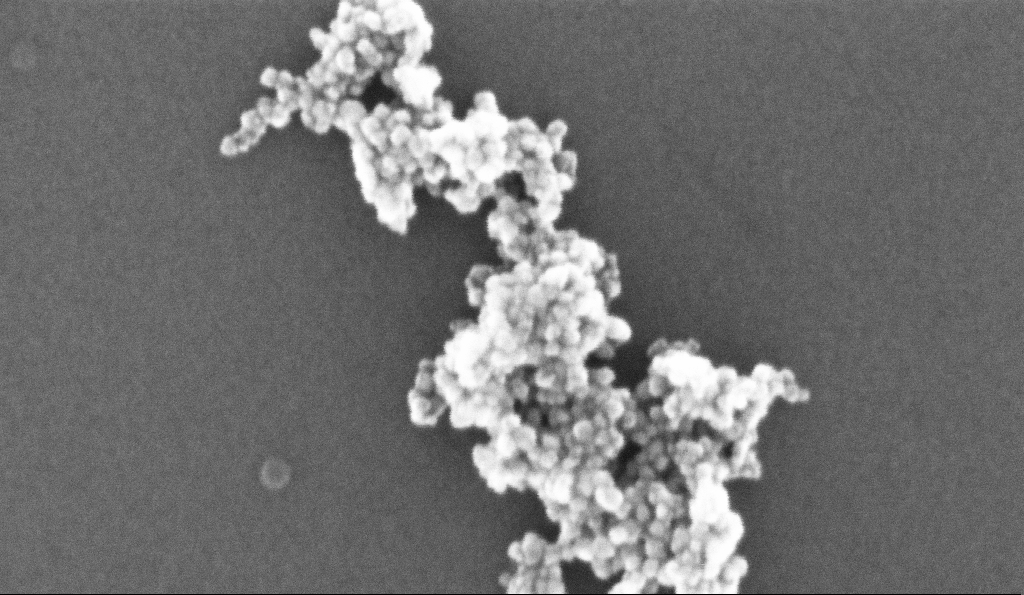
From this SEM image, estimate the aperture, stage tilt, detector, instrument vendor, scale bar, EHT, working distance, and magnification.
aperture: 30 µm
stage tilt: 0°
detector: InLens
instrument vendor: Zeiss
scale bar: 100 nm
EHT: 10 kV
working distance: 5.2 mm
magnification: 411.87 K X